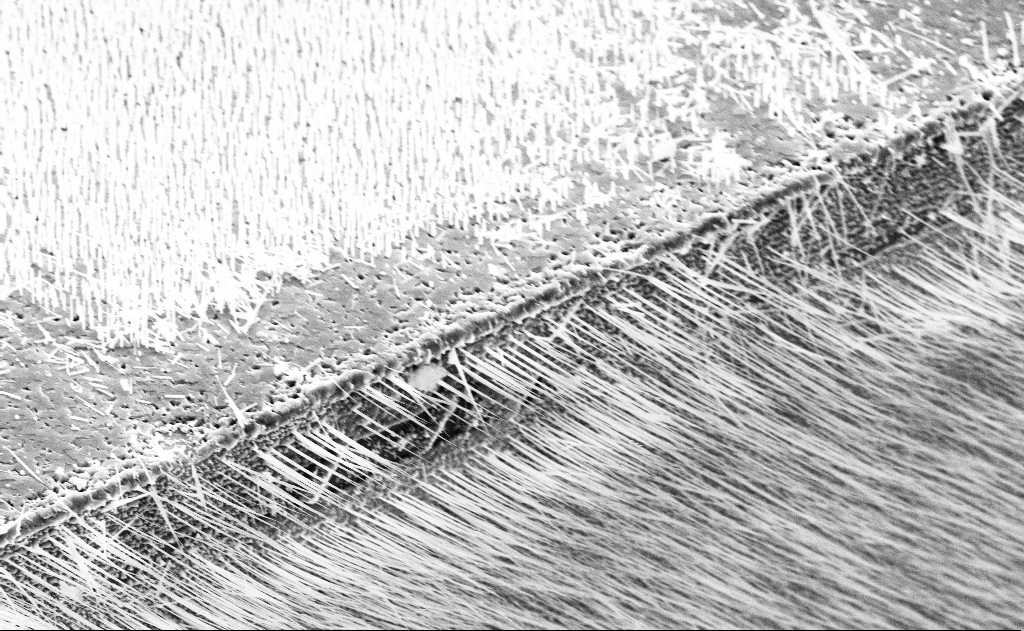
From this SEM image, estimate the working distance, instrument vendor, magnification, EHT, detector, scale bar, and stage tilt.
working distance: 14 mm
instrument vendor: Zeiss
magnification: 7.76 K X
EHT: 10 kV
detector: SE2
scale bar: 2000 nm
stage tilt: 45°